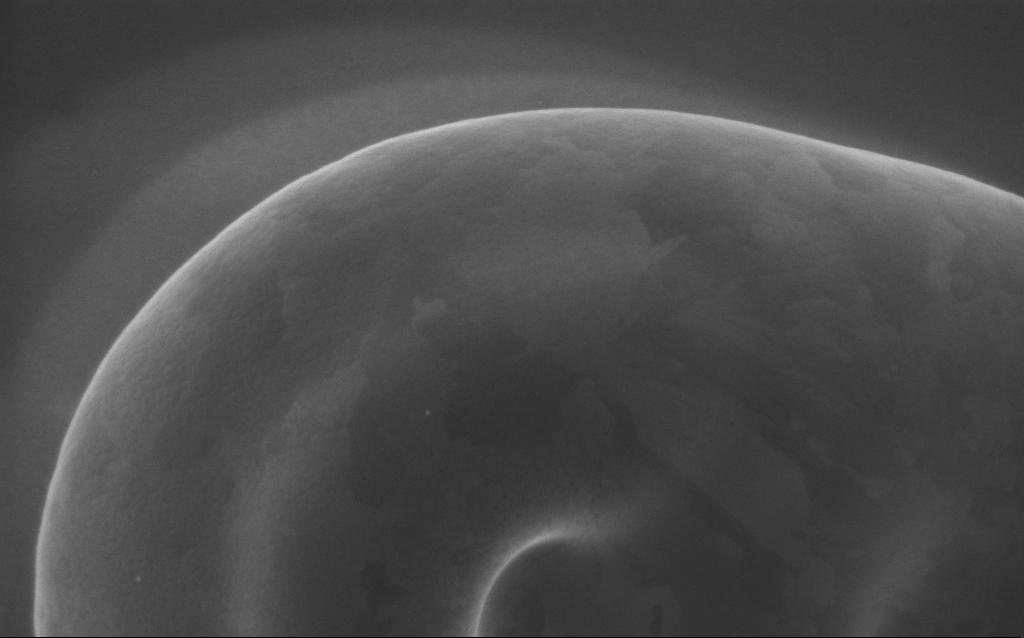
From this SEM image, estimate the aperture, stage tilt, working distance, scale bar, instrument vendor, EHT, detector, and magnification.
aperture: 30 µm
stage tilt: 0°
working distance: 3 mm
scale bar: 200 nm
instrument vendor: Zeiss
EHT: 5 kV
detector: InLens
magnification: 100 K X